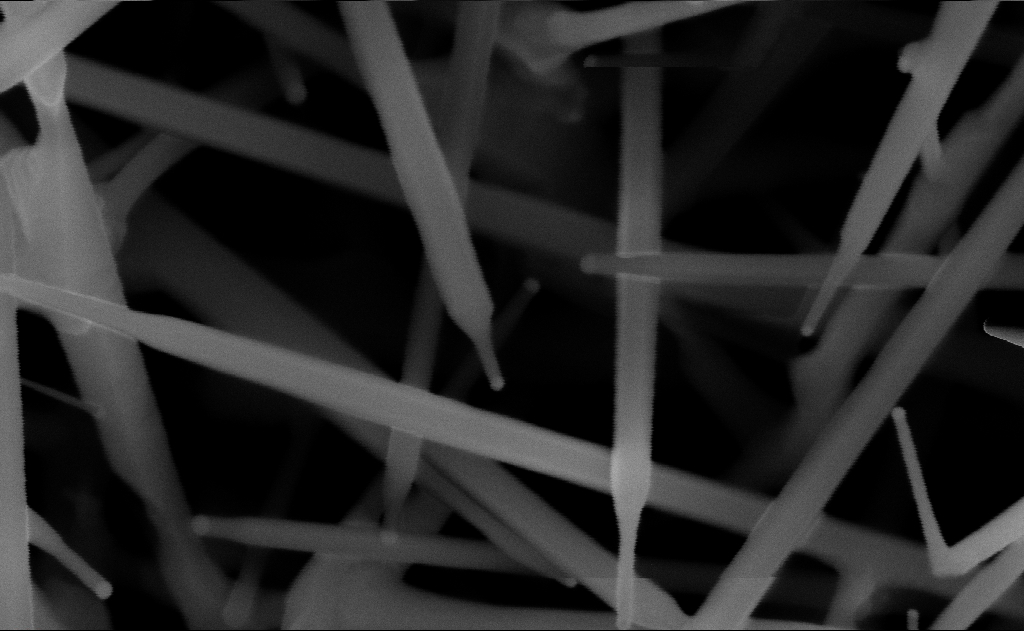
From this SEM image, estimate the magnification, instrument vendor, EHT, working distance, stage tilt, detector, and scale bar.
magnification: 200 K X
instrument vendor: Zeiss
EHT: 10 kV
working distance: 13 mm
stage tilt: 0°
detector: InLens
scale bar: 200 nm